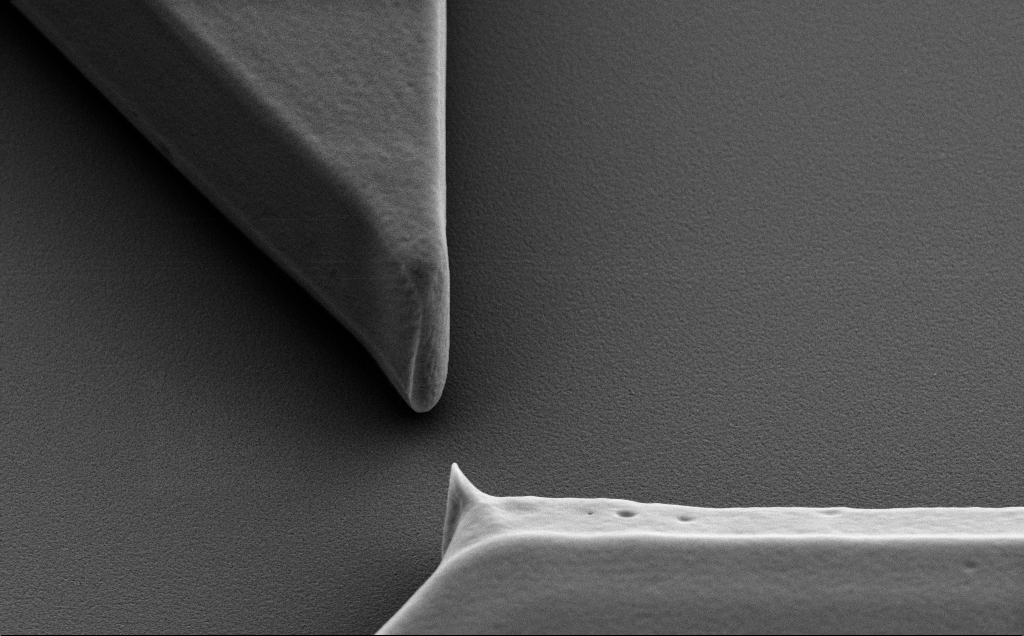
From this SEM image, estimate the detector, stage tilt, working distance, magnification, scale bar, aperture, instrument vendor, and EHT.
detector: SE2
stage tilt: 40°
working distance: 9 mm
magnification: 15 K X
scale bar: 1000 nm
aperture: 30 µm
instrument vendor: Zeiss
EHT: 5 kV